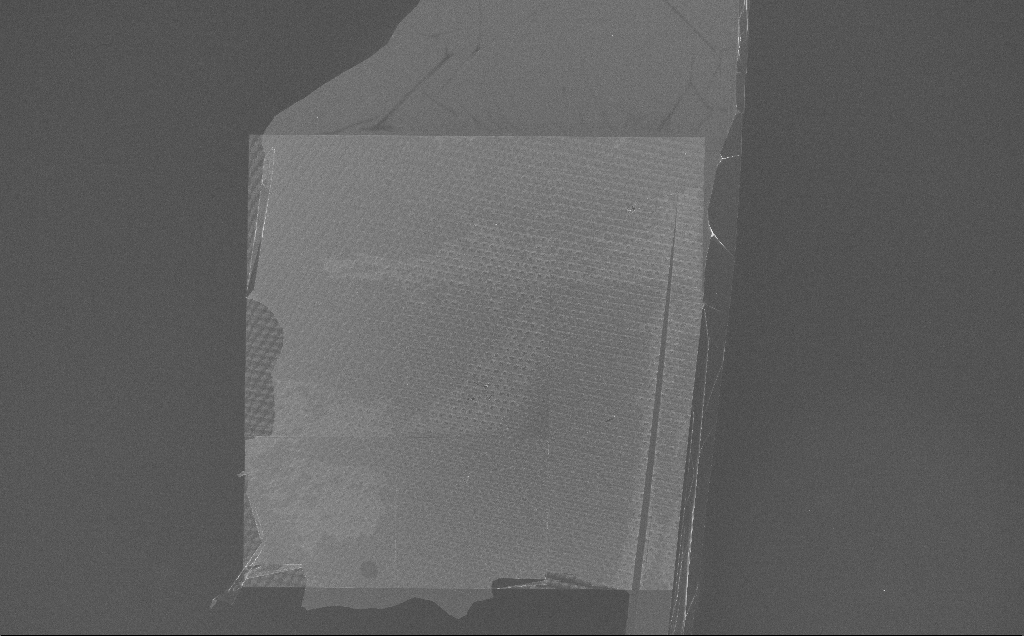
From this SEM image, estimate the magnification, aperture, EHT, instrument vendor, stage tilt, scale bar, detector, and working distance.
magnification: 0.225 K X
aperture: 30 µm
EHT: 10 kV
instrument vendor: Zeiss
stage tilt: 0°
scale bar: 100000 nm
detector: InLens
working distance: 7 mm